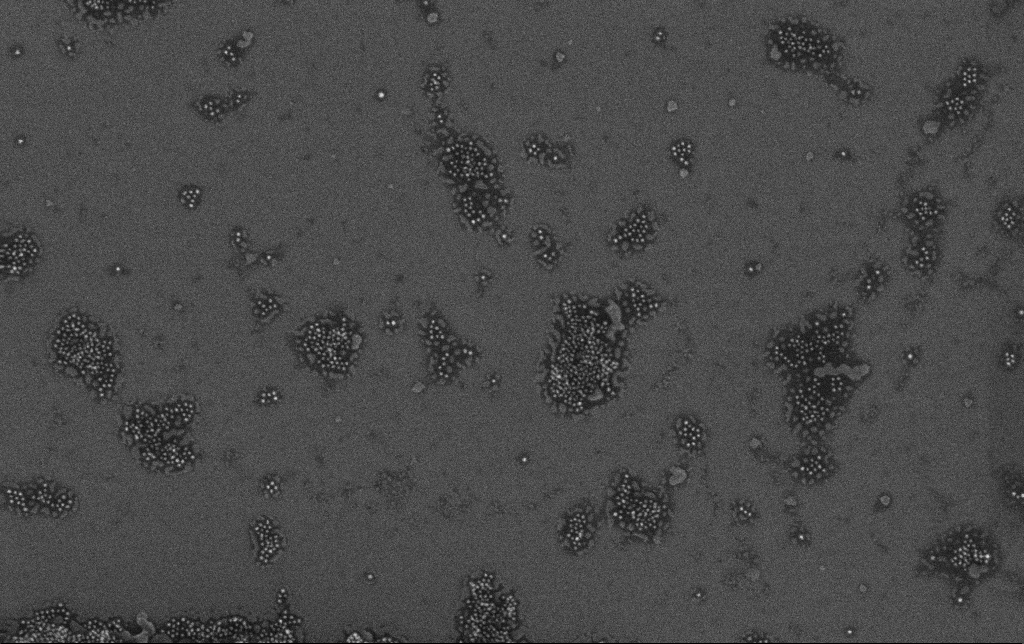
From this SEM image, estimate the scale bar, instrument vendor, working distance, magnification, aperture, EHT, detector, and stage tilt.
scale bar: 200 nm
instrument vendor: Zeiss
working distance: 3.4 mm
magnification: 100 K X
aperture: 30 µm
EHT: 10 kV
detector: InLens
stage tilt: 0°